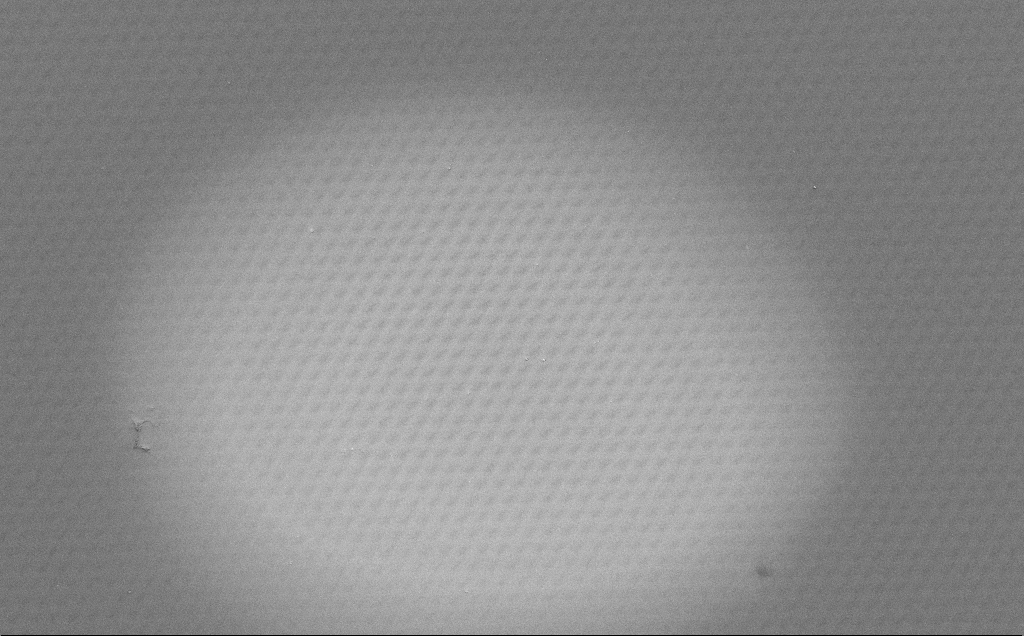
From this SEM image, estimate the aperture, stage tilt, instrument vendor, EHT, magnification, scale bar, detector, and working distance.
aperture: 30 µm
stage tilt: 0°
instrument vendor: Zeiss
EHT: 1.5 kV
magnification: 0.698 K X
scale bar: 100000 nm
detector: SE2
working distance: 7 mm